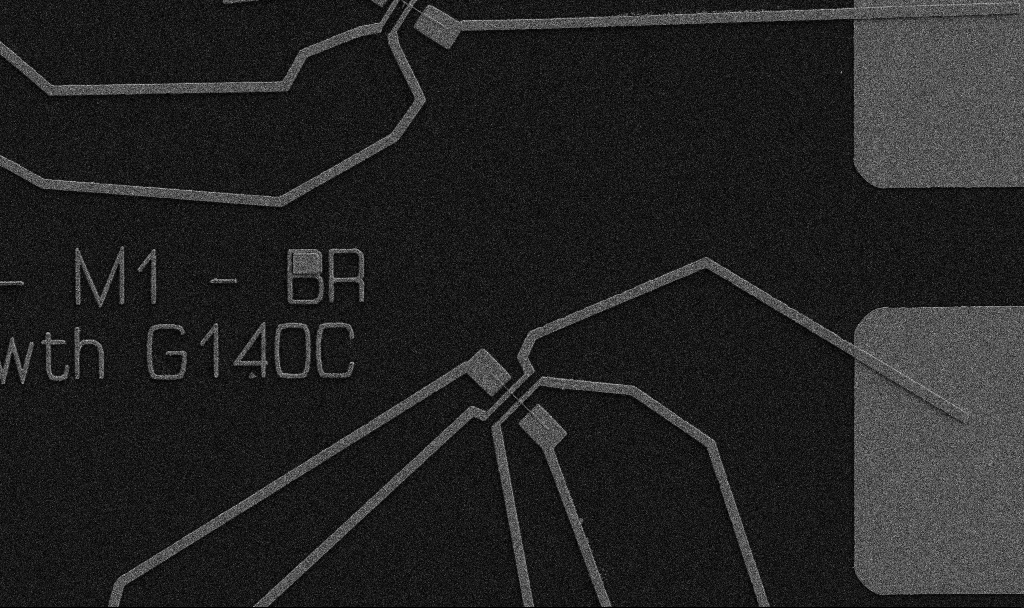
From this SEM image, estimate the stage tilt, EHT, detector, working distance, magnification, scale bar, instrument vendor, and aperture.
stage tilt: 0°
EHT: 5 kV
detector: SE2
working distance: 10.7 mm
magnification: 5 K X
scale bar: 10000 nm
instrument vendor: Zeiss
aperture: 30 µm